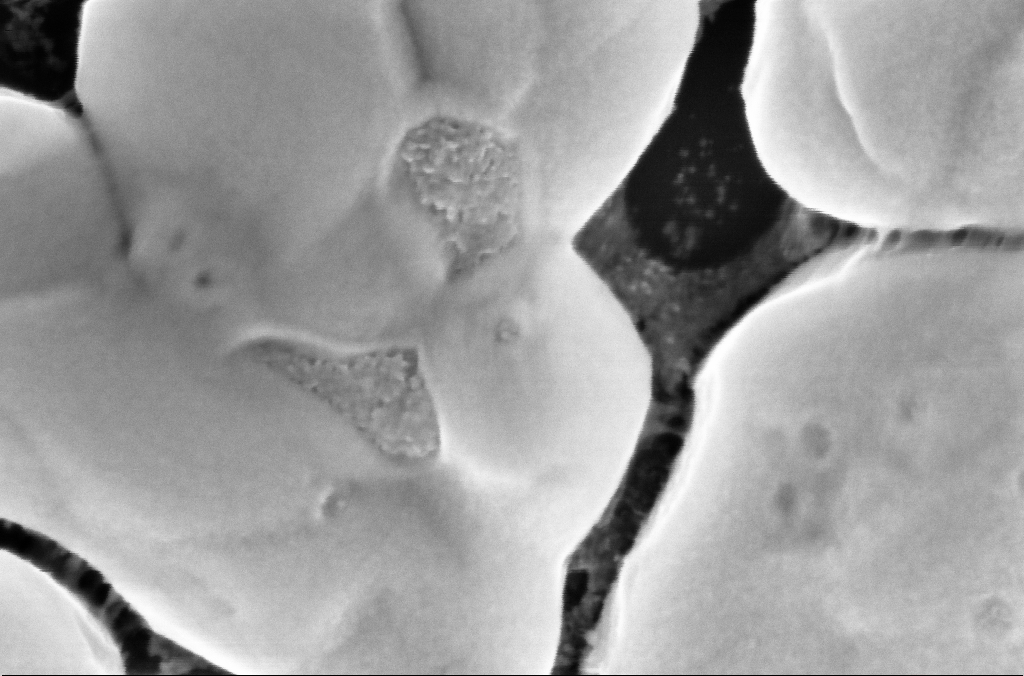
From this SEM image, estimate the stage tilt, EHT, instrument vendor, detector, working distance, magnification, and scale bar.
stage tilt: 0°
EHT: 5 kV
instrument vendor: Zeiss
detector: InLens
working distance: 3.1 mm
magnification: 300 K X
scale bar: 100 nm